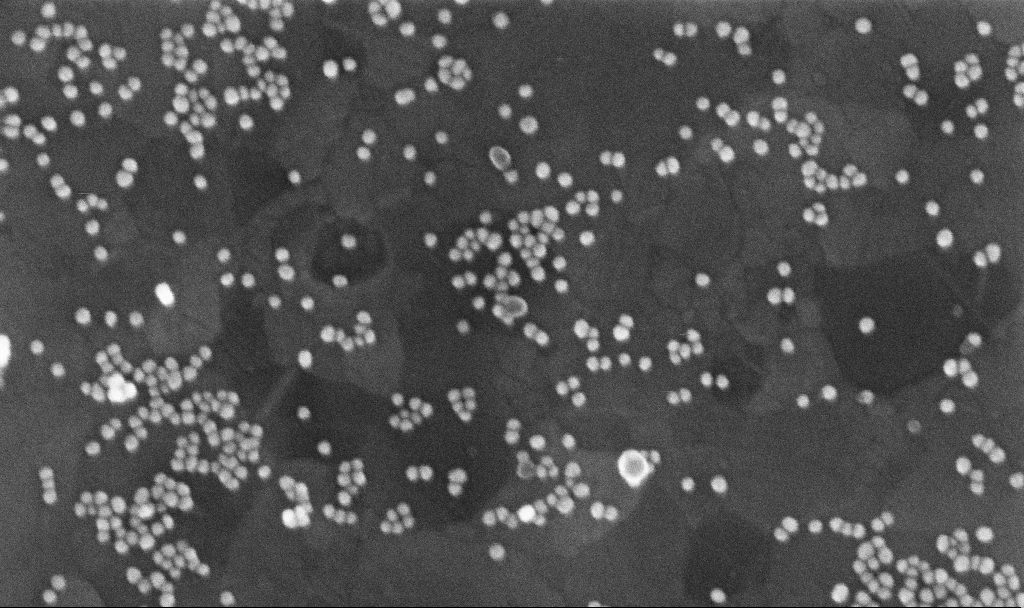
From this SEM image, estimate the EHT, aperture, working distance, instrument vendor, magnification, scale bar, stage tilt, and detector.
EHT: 10 kV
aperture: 30 µm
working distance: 3.7 mm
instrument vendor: Zeiss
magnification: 266.66 K X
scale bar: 200 nm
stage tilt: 0°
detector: InLens